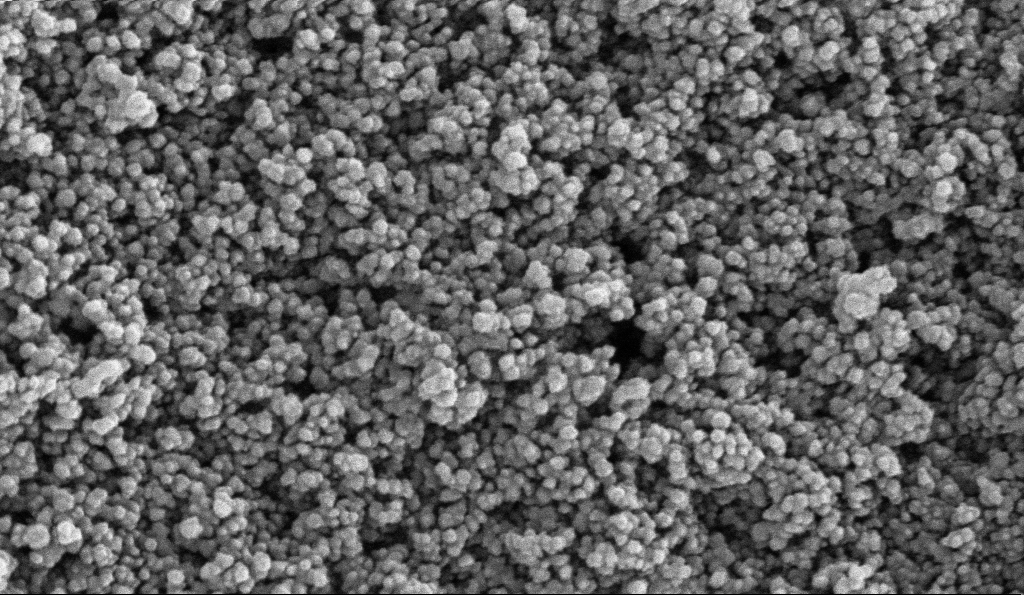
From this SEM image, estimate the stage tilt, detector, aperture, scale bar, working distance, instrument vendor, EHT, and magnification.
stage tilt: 0°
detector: InLens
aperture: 30 µm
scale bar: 100 nm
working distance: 5.1 mm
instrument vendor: Zeiss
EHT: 10 kV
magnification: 135 K X